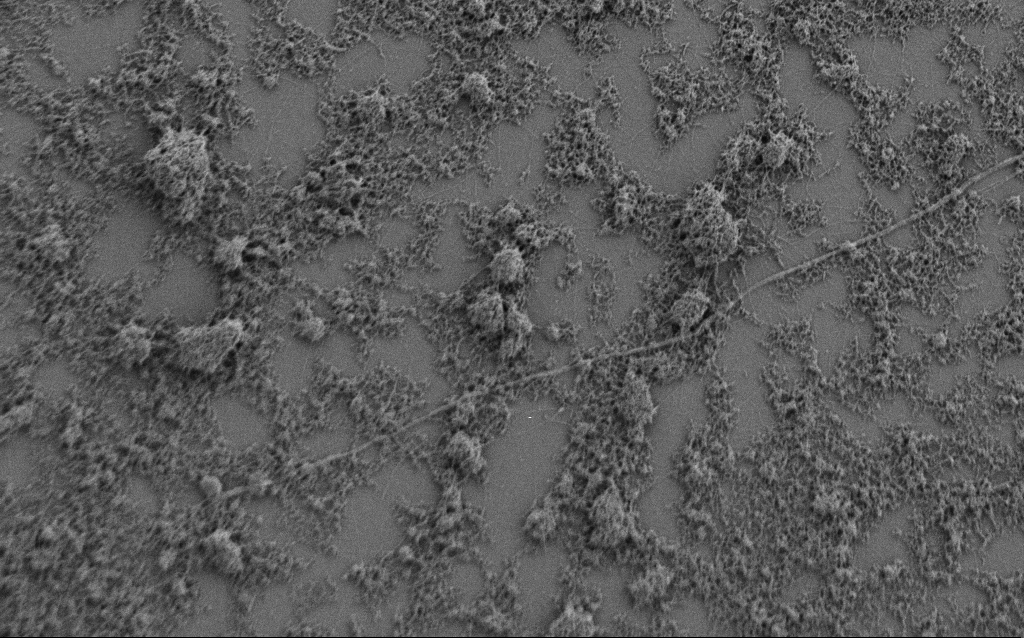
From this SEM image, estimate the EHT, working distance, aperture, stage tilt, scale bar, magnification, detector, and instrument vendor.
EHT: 0.9 kV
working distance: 7 mm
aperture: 30 µm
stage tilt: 0°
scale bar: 2000 nm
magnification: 7.5 K X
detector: SE2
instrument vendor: Zeiss